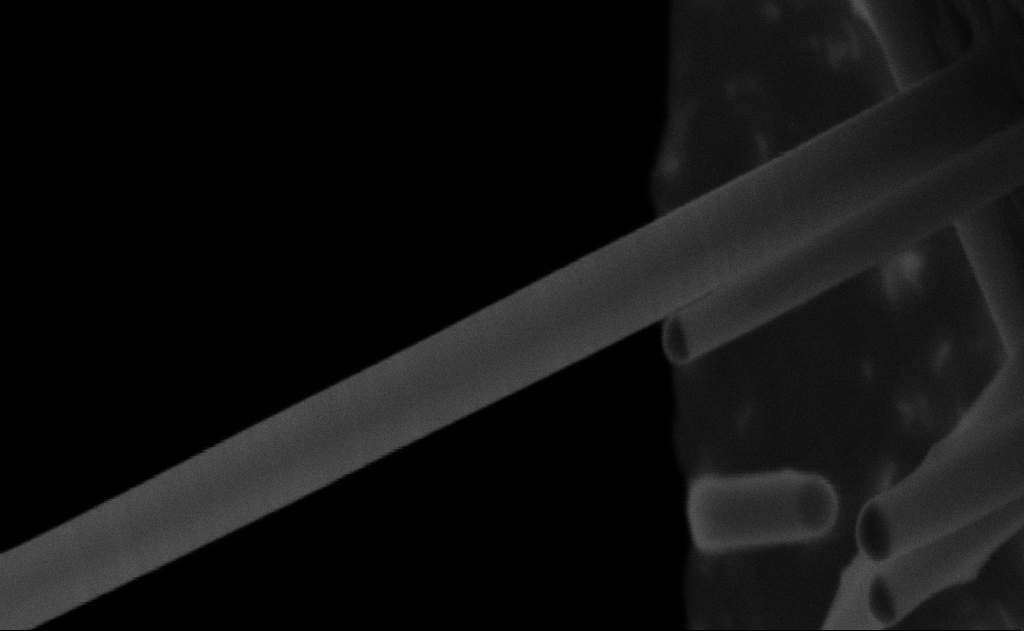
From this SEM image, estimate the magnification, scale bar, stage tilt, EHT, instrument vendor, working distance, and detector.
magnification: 289.19 K X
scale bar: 100 nm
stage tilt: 0°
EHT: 20 kV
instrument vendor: Zeiss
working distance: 9 mm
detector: SE2